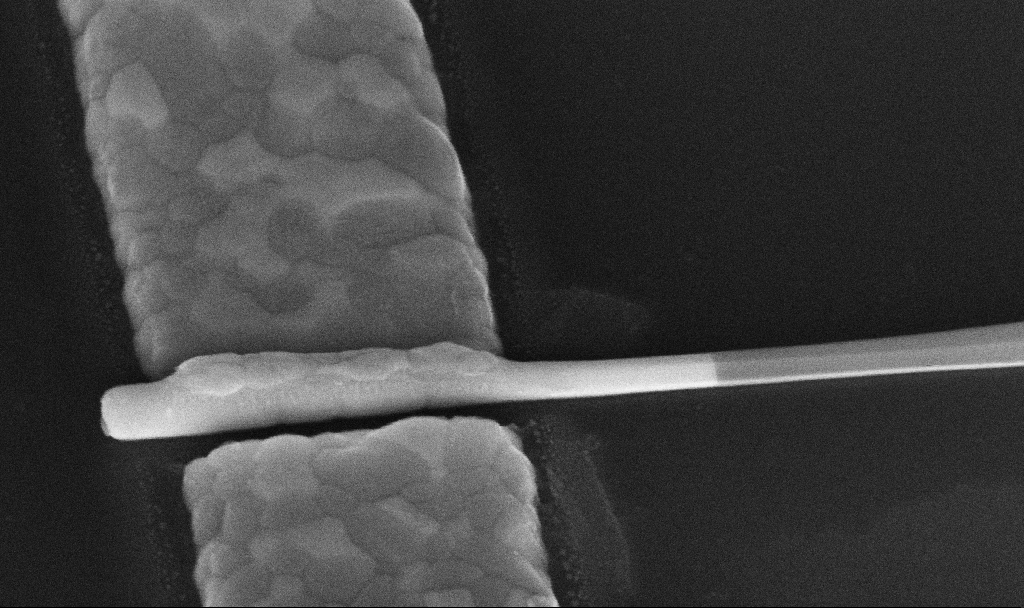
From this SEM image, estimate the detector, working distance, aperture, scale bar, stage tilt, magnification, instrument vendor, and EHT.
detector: InLens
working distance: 8.6 mm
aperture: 30 µm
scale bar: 200 nm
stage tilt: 45°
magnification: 182.03 K X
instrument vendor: Zeiss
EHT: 10 kV